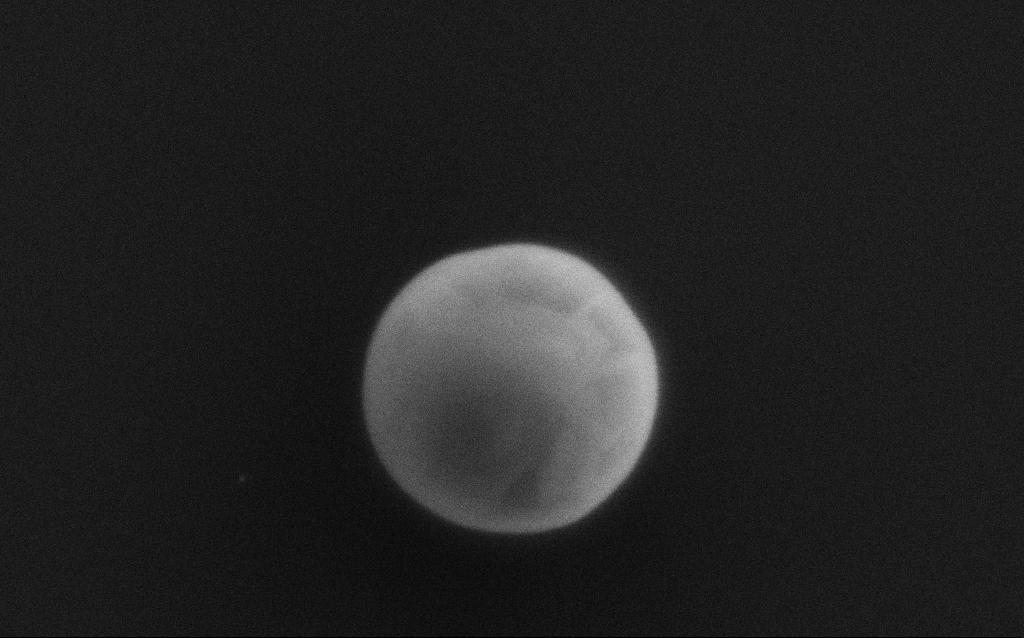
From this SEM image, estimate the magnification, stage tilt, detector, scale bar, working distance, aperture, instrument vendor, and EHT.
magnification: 168.7 K X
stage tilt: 0°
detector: SE2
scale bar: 200 nm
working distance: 3 mm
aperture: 30 µm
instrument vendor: Zeiss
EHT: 2 kV